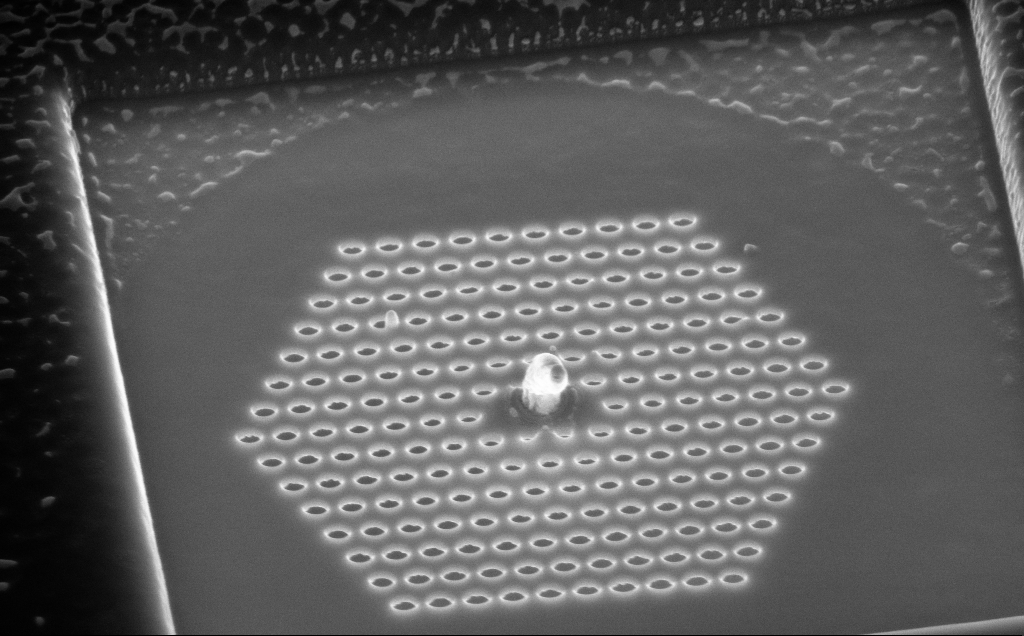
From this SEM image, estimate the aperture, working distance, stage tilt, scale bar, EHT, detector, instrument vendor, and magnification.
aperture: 30 µm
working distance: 8 mm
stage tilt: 45°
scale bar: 1000 nm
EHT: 10 kV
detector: InLens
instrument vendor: Zeiss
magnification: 54.71 K X